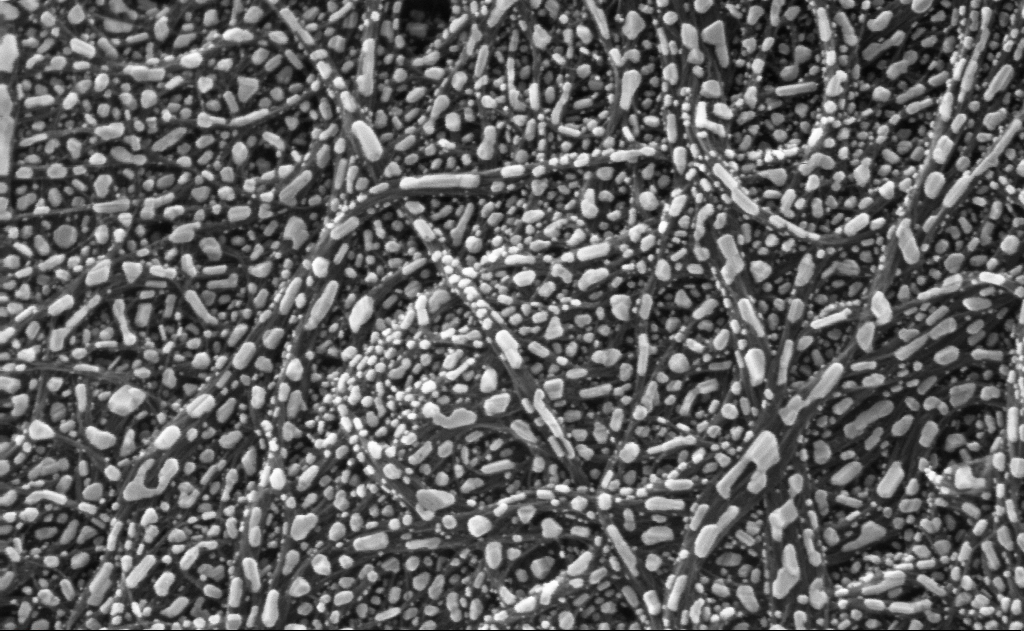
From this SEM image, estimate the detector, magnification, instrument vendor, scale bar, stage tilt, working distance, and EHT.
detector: InLens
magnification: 278.98 K X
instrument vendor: Zeiss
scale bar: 200 nm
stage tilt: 0°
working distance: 3 mm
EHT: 10 kV